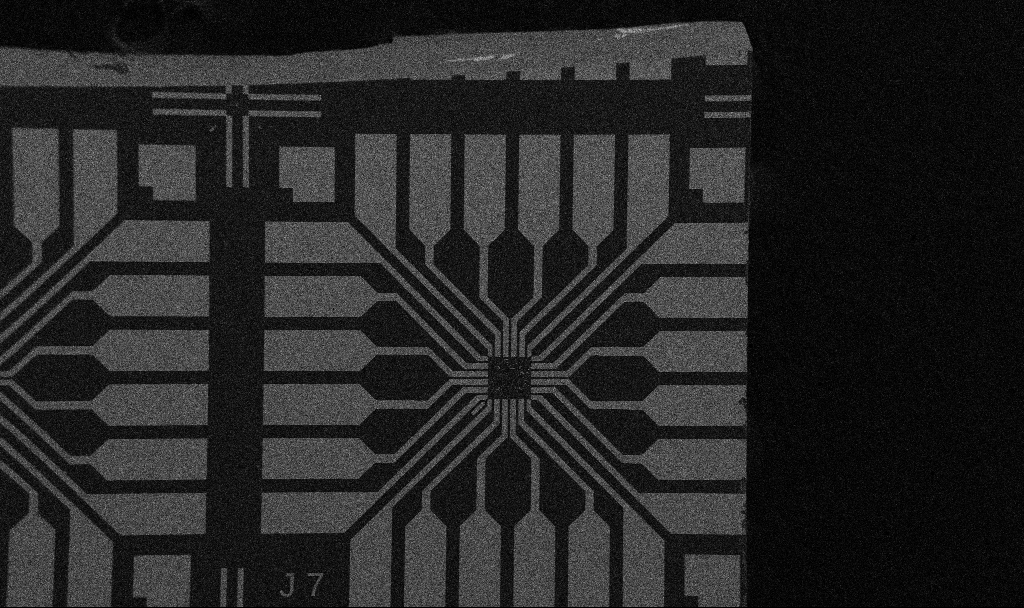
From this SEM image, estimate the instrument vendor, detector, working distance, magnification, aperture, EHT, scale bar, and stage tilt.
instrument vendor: Zeiss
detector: SE2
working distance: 10.7 mm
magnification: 0.1 K X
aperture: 30 µm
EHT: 5 kV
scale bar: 200000 nm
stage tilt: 0°